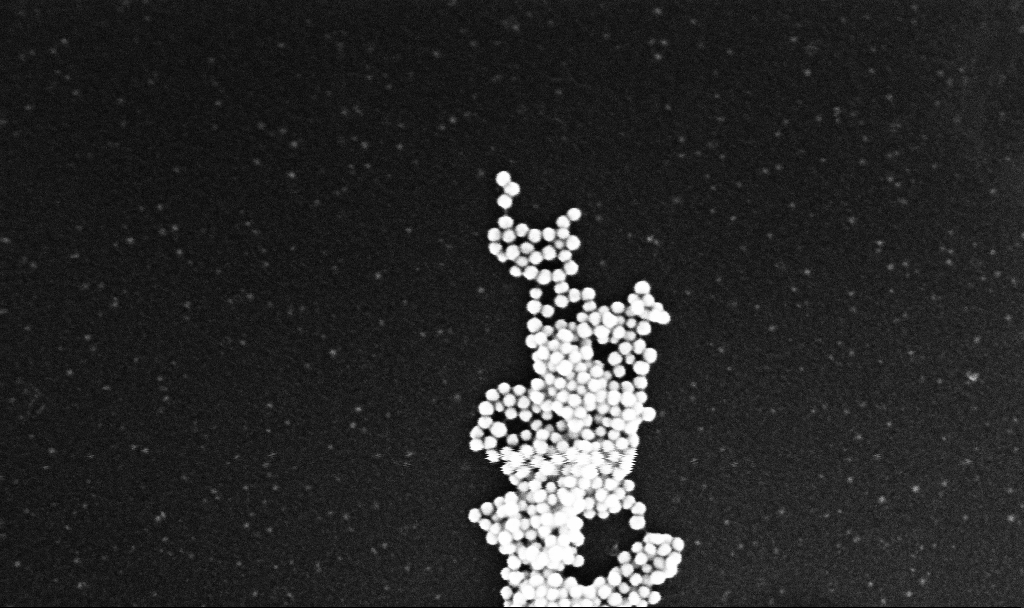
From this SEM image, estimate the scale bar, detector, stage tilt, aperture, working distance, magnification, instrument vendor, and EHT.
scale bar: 200 nm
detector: InLens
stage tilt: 0°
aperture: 30 µm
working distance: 3.2 mm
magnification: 223.63 K X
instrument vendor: Zeiss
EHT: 10 kV